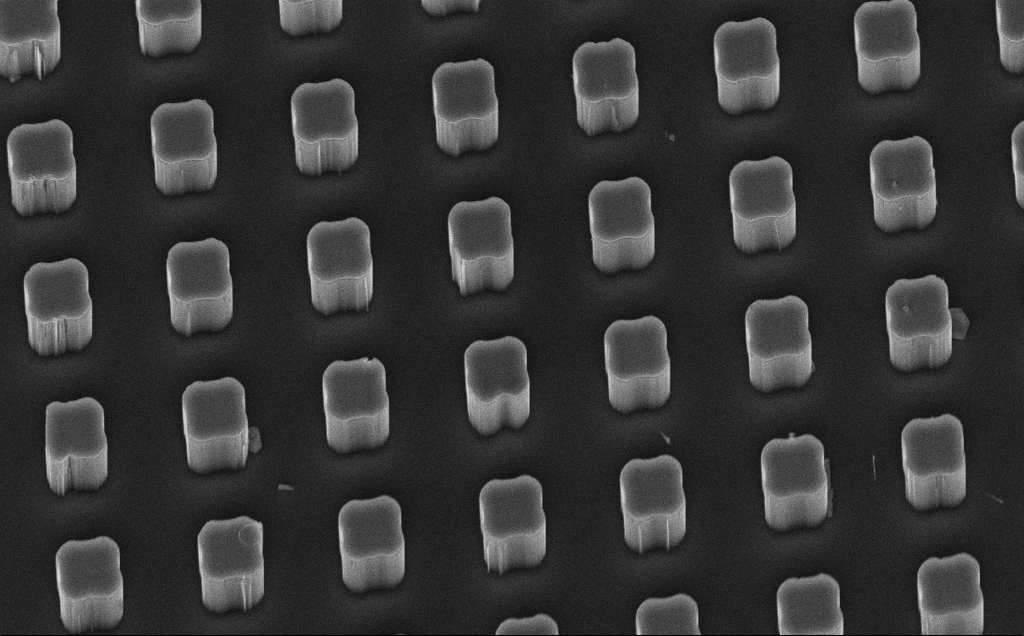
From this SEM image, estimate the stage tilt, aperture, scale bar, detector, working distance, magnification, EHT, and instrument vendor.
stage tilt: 45°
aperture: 30 µm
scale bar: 10000 nm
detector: InLens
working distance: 11 mm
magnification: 5.2 K X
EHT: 10 kV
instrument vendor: Zeiss